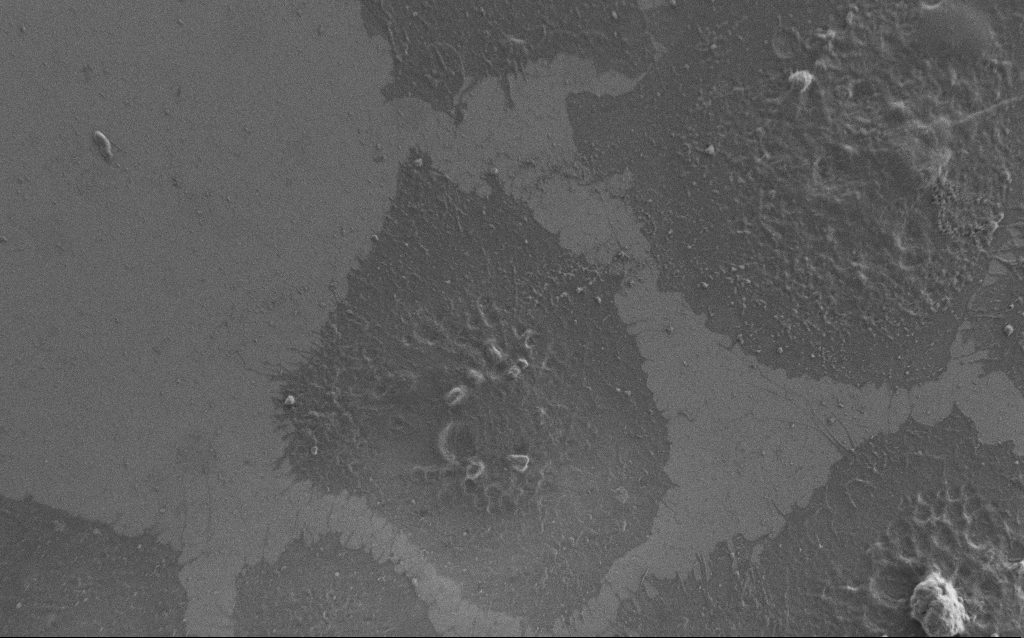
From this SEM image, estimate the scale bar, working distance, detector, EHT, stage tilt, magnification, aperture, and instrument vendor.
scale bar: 10000 nm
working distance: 6 mm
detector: SE2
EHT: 1 kV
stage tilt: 0°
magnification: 3 K X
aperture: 30 µm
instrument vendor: Zeiss